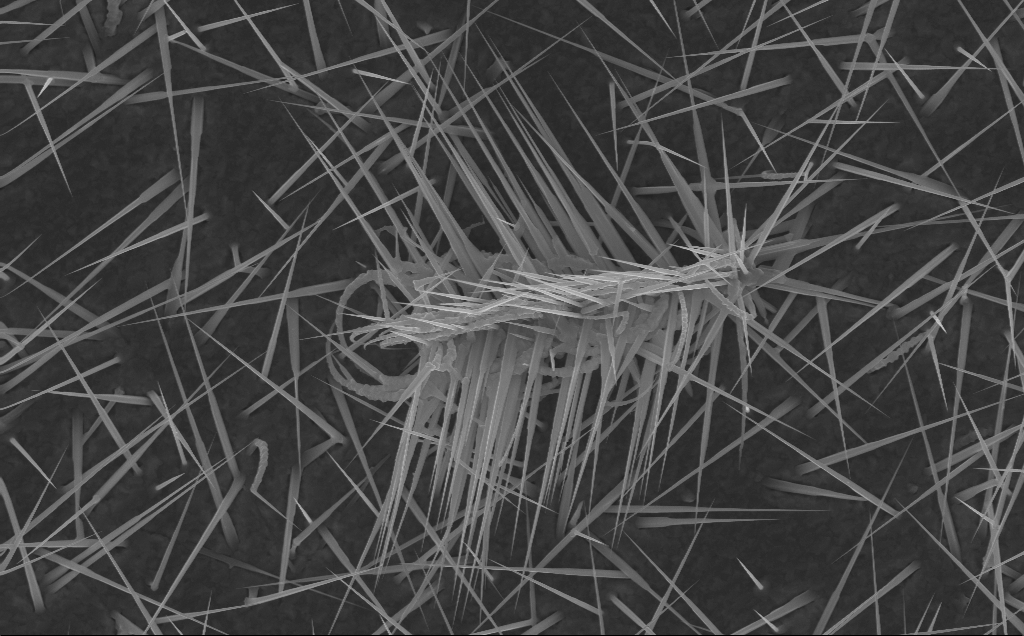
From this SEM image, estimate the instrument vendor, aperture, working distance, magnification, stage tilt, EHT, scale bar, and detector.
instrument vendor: Zeiss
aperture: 30 µm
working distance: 6 mm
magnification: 20.24 K X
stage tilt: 0°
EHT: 10 kV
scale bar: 2000 nm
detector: InLens